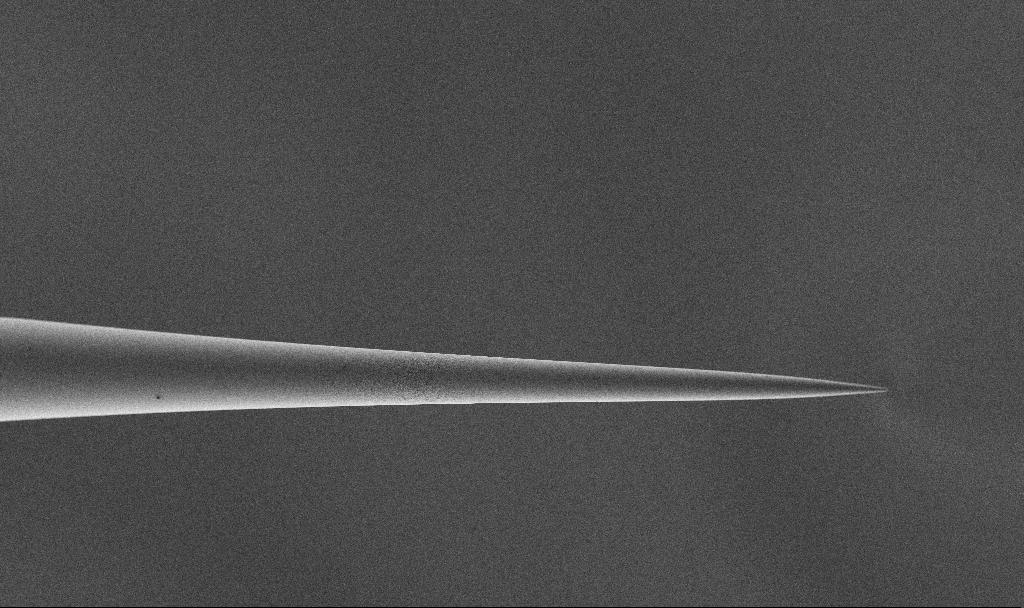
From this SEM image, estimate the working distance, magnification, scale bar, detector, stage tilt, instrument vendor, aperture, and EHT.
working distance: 3.3 mm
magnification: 1.34 K X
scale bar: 10000 nm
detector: SE2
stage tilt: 45°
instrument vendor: Zeiss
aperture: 30 µm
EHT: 1.5 kV